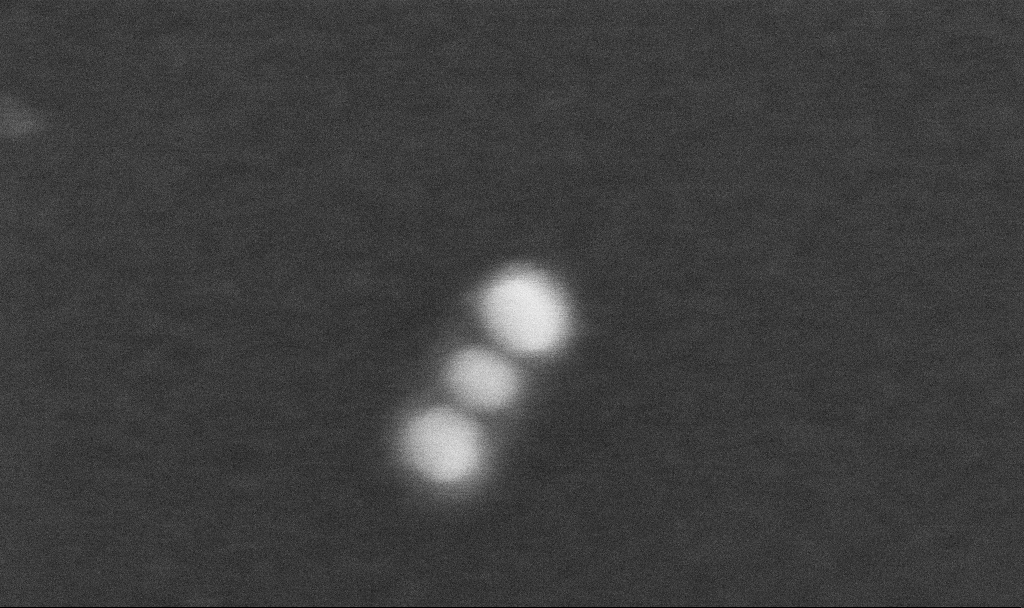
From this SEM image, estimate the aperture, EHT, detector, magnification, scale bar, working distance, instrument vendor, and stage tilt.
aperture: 30 µm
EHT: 10 kV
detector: InLens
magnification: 1299.6 K X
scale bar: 20 nm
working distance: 3.3 mm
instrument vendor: Zeiss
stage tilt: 0°